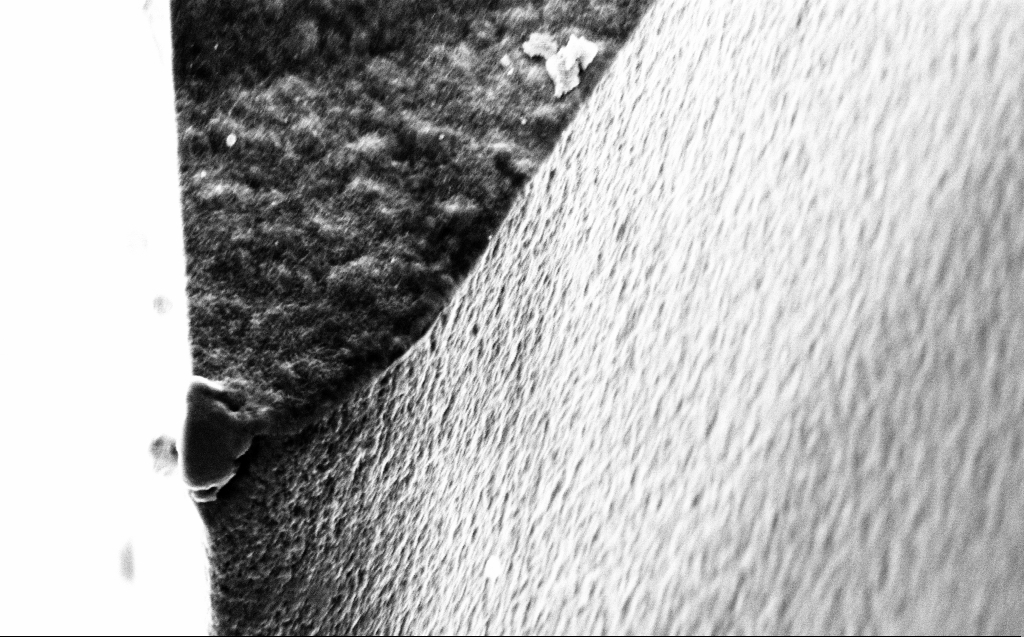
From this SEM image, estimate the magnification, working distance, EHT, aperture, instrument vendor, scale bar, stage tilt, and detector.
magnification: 39.97 K X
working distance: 5 mm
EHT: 5 kV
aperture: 30 µm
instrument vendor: Zeiss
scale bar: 1000 nm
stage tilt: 45°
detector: InLens